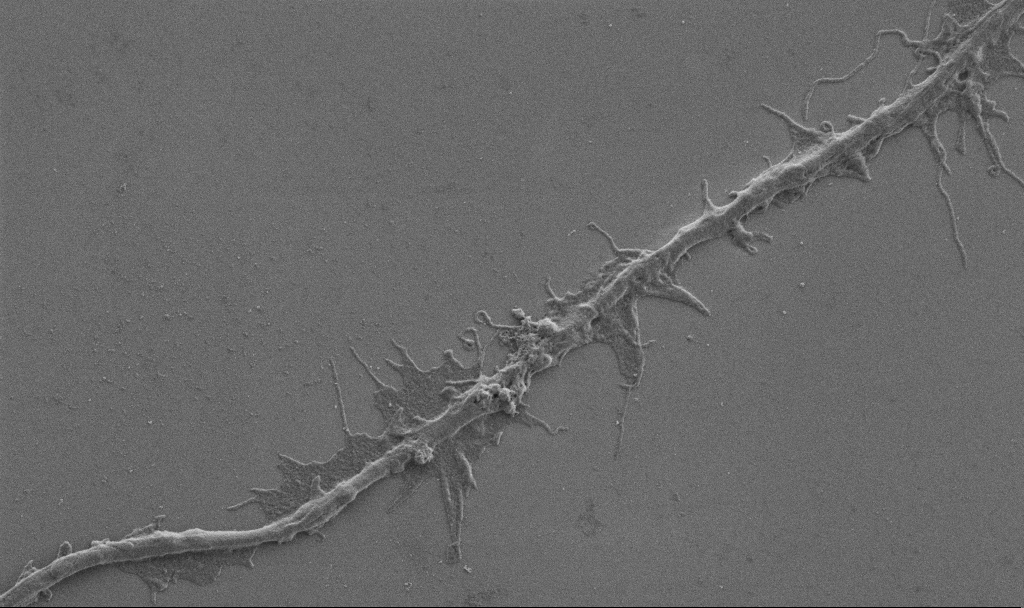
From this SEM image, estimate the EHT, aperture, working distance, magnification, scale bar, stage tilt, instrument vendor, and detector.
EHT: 1 kV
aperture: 30 µm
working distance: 6.9 mm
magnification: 10 K X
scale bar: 2000 nm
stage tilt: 0°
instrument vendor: Zeiss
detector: SE2